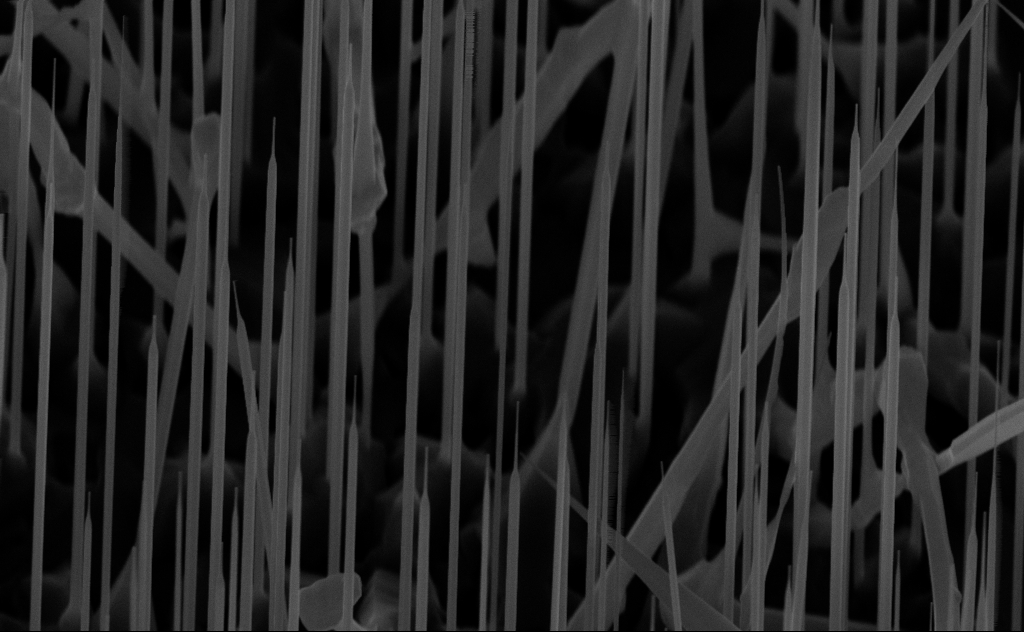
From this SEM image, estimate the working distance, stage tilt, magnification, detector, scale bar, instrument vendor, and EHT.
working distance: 7 mm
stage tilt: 44.9°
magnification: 40 K X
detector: InLens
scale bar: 1000 nm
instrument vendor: Zeiss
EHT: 10 kV